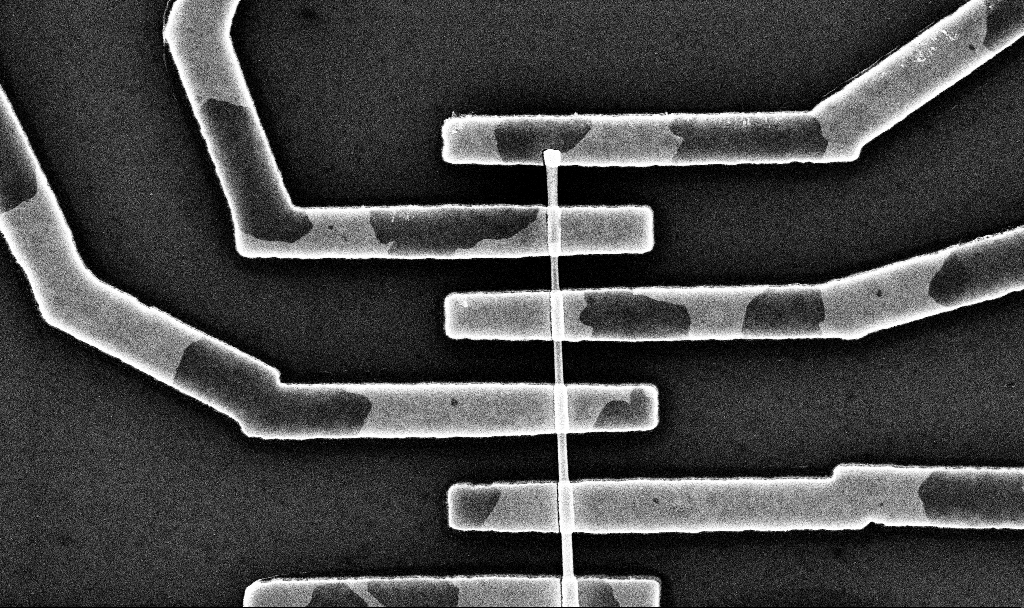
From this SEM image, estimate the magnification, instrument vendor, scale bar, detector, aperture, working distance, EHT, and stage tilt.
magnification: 30.23 K X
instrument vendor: Zeiss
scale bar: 1000 nm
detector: InLens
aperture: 30 µm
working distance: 6.8 mm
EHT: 10 kV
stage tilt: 0°